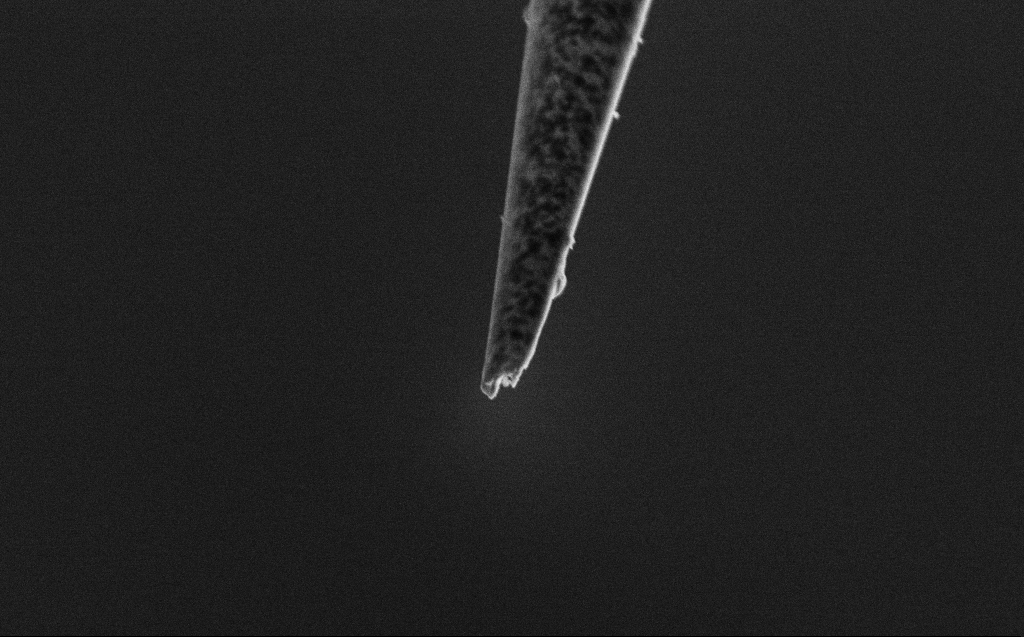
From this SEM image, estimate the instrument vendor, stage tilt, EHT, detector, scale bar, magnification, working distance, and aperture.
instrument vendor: Zeiss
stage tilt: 45°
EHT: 2 kV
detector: SE2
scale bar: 1000 nm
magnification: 50 K X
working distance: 4 mm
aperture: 30 µm